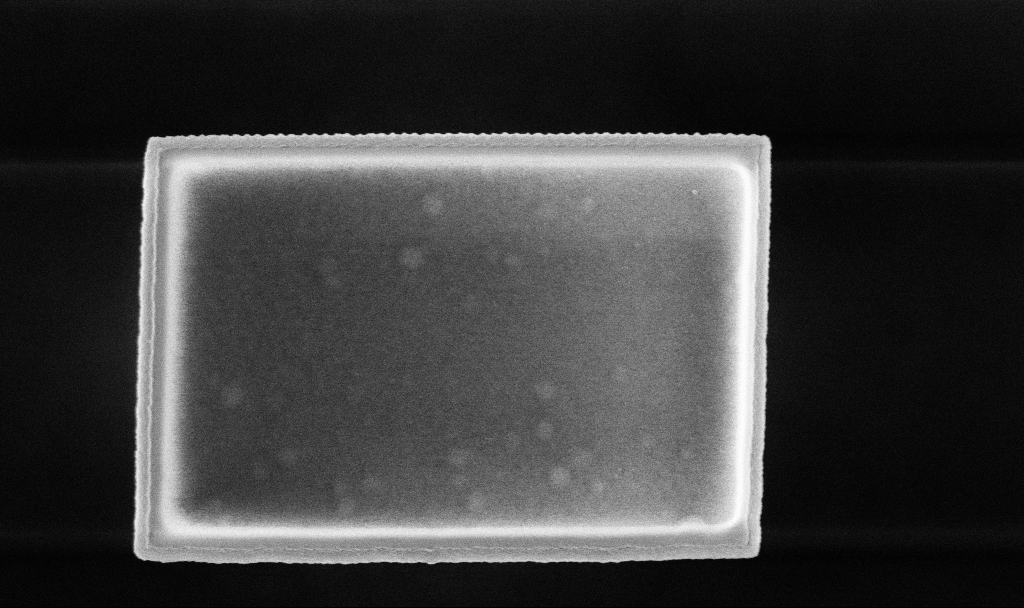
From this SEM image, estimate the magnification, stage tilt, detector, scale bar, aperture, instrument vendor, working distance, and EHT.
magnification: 53.04 K X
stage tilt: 0°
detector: InLens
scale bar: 1000 nm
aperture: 30 µm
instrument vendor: Zeiss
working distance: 3.3 mm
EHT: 5 kV